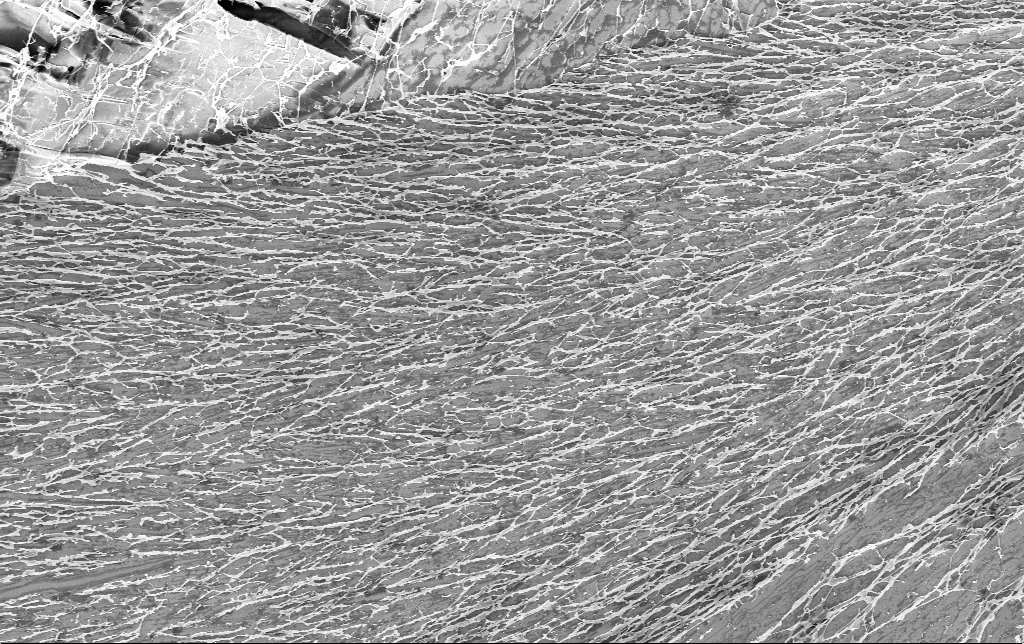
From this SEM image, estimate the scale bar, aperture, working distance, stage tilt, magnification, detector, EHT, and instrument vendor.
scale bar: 10000 nm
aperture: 30 µm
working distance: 3.4 mm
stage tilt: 0°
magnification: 3.48 K X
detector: InLens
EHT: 3 kV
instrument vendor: Zeiss